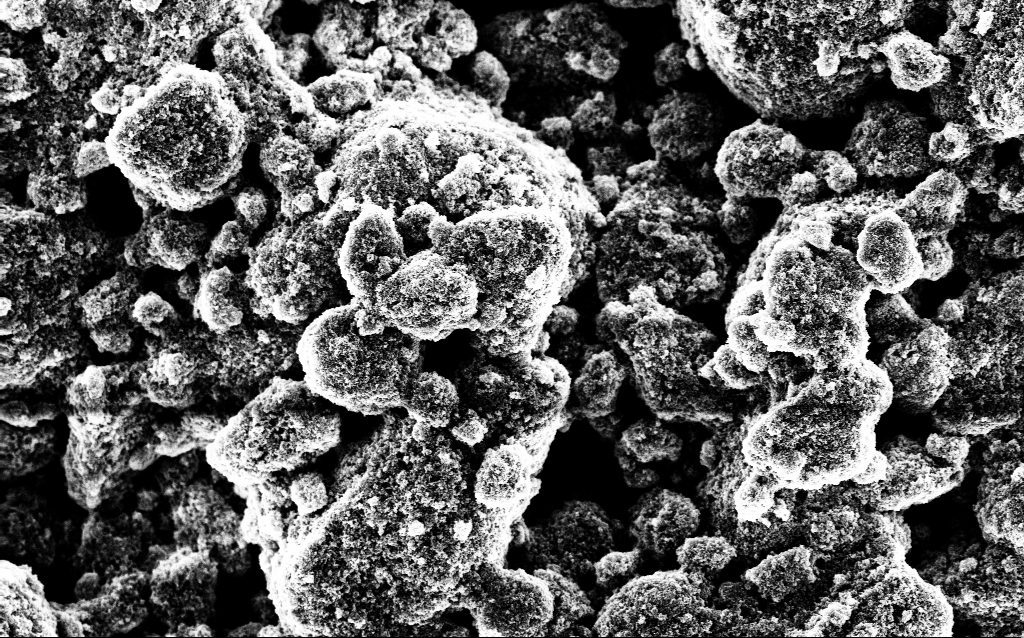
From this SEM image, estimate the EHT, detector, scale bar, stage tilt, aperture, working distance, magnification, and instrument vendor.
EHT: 5 kV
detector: InLens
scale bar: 2000 nm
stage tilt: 0°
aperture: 30 µm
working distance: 1.8 mm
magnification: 14.15 K X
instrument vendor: Zeiss